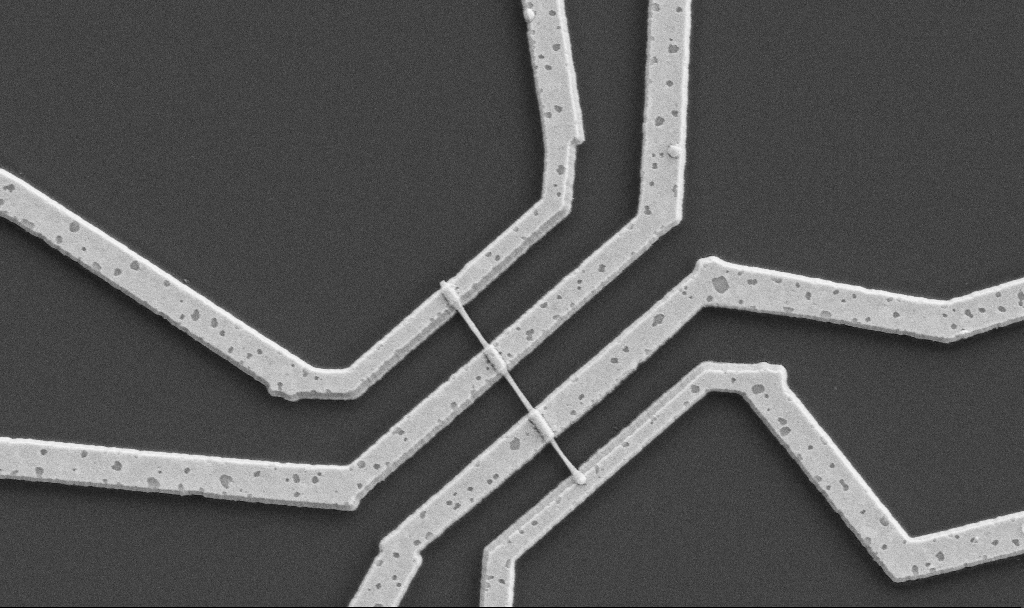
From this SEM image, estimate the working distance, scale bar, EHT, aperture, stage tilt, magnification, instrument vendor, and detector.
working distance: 10.7 mm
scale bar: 1000 nm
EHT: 5 kV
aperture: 30 µm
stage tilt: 0°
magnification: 20 K X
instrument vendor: Zeiss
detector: SE2